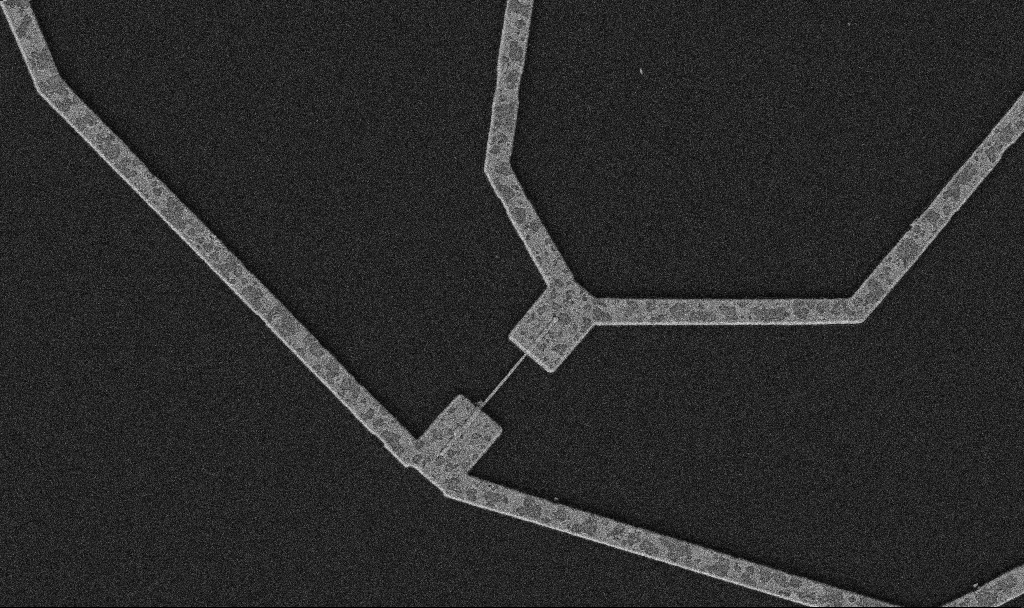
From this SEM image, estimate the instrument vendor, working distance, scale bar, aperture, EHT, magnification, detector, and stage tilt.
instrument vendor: Zeiss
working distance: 10.7 mm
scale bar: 2000 nm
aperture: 30 µm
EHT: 5 kV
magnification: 10 K X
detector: SE2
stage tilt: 0°